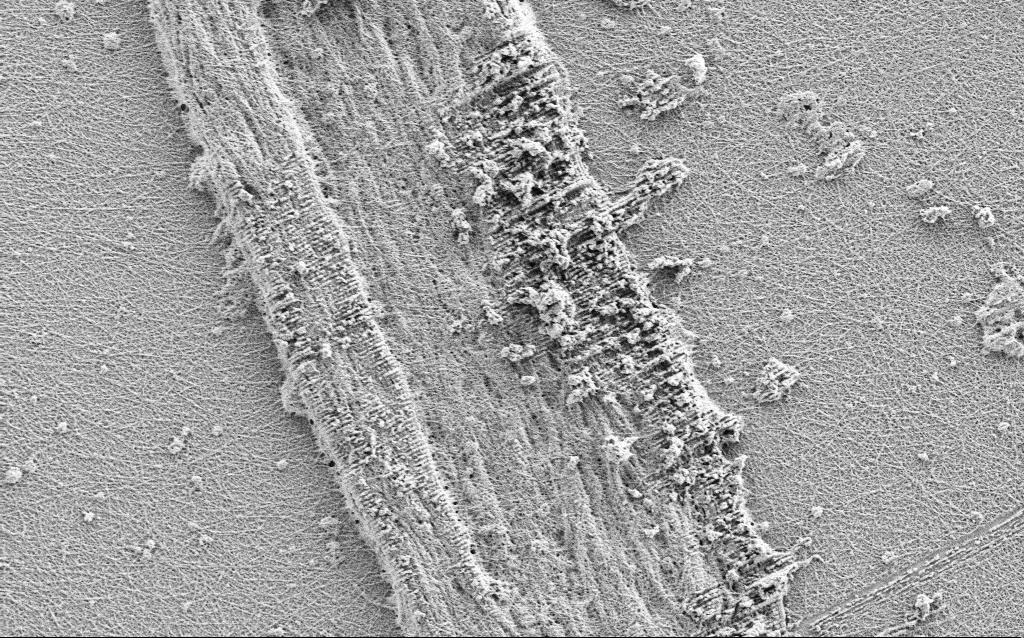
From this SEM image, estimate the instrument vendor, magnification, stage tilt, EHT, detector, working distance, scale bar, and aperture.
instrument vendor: Zeiss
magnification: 10 K X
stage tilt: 0°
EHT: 0.9 kV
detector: SE2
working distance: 3 mm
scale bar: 2000 nm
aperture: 30 µm